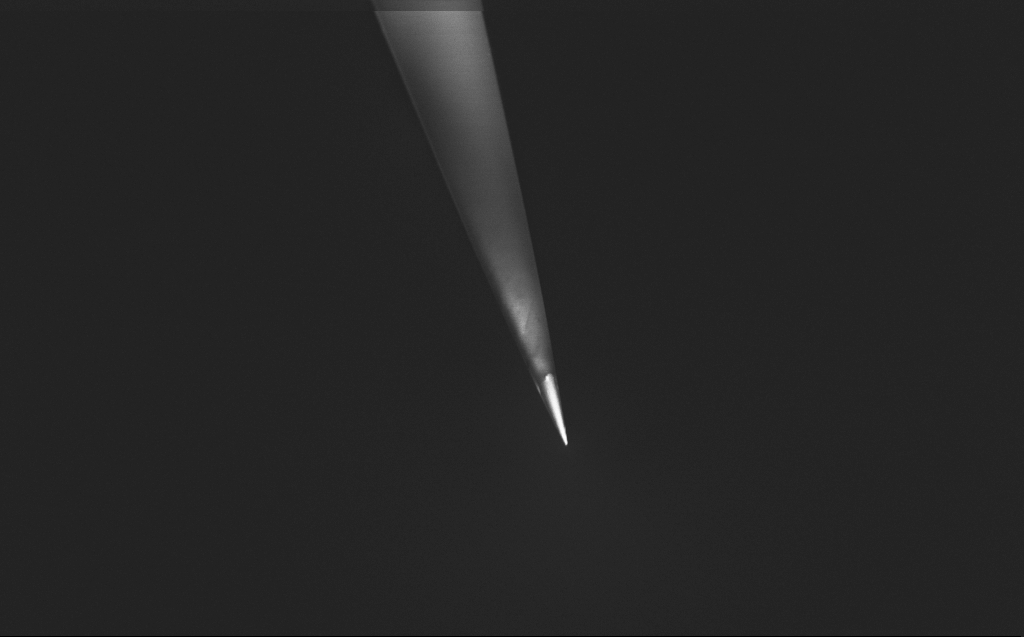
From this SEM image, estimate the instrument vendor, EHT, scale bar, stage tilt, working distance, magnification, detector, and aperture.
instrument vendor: Zeiss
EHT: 0.8 kV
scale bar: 2000 nm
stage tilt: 39.3°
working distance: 5 mm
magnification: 10 K X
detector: InLens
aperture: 30 µm